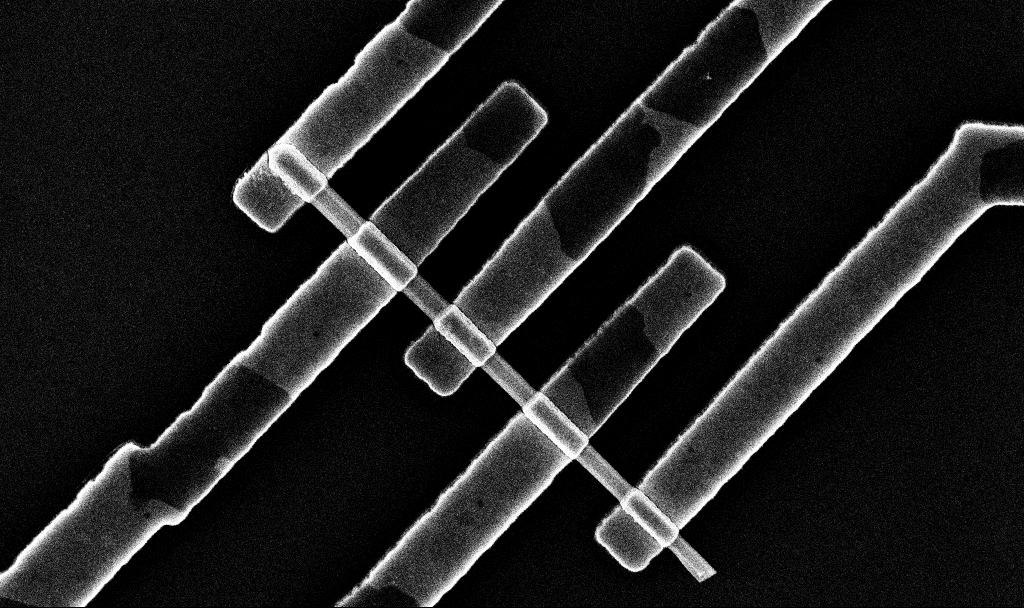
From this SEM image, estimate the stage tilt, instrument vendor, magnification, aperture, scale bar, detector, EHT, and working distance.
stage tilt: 0°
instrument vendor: Zeiss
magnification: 40.64 K X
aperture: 30 µm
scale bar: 1000 nm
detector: InLens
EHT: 10 kV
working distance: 6.7 mm